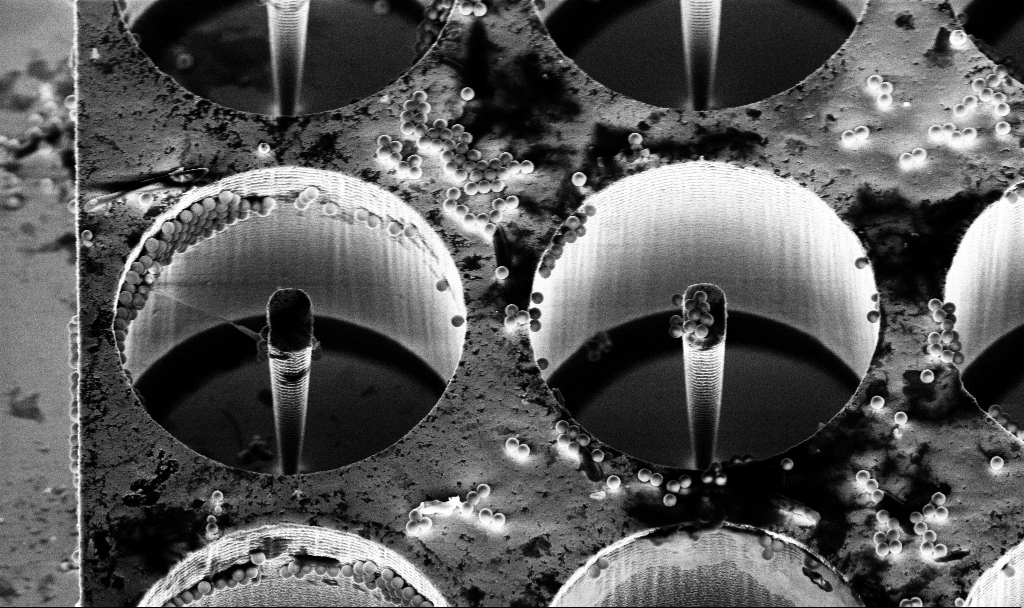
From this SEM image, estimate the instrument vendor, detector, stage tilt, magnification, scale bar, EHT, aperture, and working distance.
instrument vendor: Zeiss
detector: InLens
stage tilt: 30°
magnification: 6.61 K X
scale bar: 10000 nm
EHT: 5 kV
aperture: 30 µm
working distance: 7.1 mm